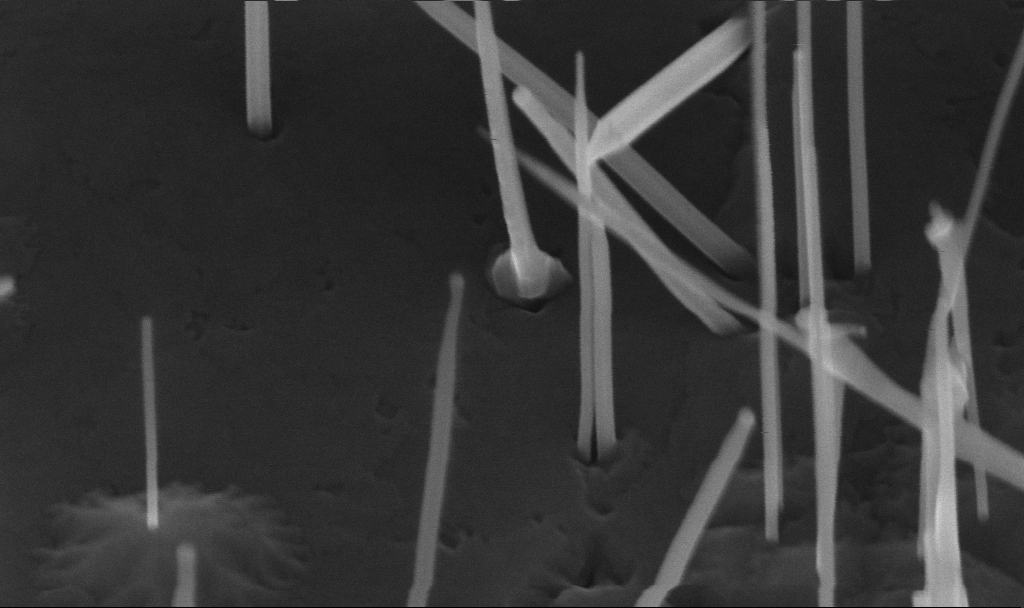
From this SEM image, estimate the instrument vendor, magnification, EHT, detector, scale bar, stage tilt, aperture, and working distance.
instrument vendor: Zeiss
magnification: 209.21 K X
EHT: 10 kV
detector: InLens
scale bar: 200 nm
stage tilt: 45°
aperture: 30 µm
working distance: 5.6 mm